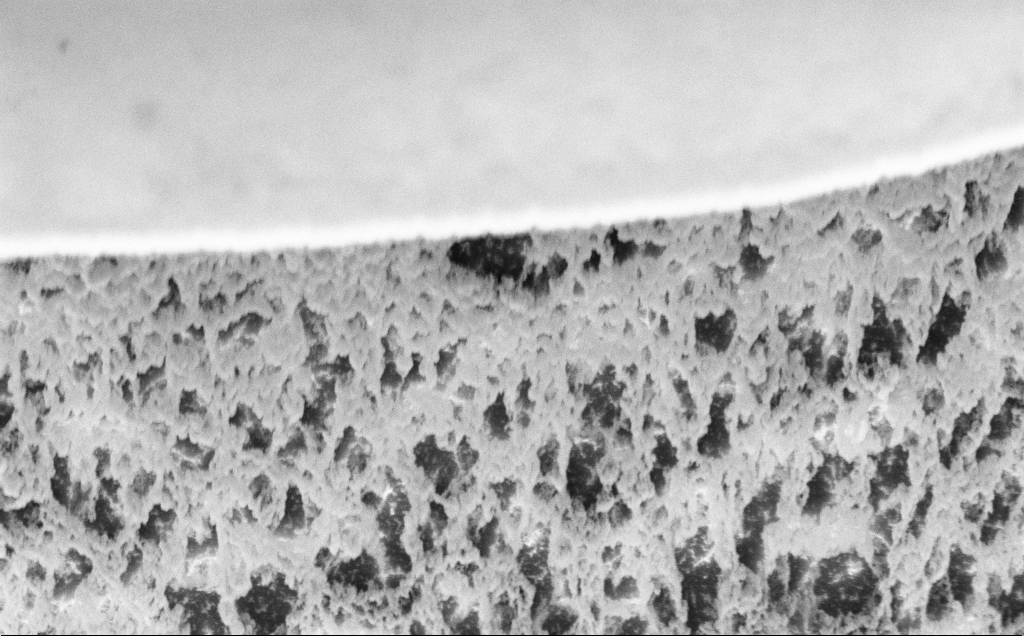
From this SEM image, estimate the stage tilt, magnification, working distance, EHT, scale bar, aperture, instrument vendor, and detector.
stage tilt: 45°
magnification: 33.68 K X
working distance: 8 mm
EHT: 5 kV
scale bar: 2000 nm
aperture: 30 µm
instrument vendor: Zeiss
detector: InLens